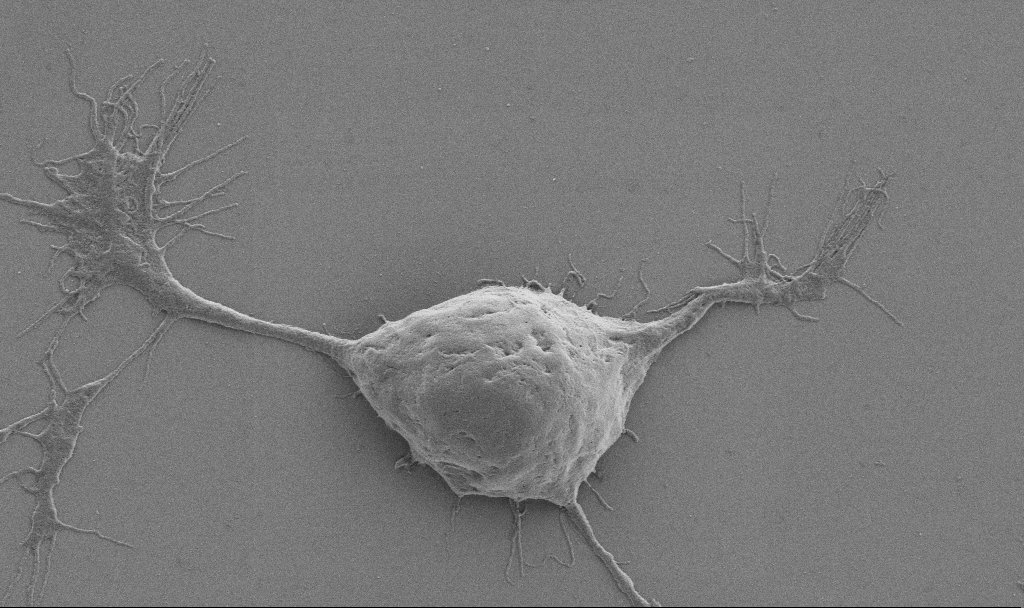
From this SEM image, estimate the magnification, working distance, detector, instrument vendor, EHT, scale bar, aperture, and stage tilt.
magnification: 10 K X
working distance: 6.9 mm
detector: SE2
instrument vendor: Zeiss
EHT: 0.9 kV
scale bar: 2000 nm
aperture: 30 µm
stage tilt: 0°